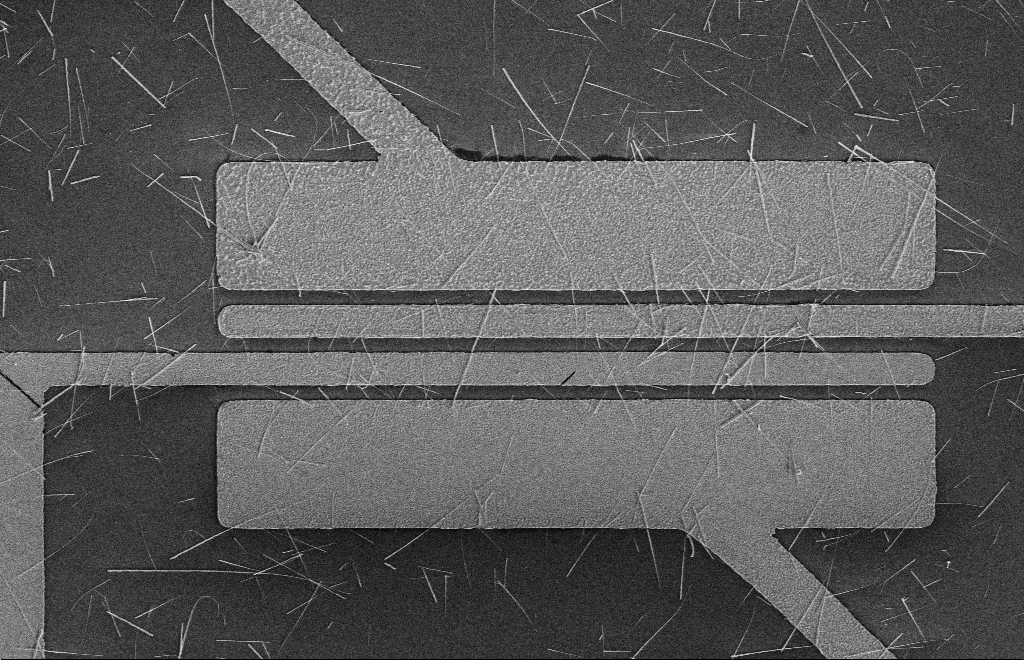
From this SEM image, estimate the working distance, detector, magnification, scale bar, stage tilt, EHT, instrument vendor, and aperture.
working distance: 16 mm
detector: SE2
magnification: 4.34 K X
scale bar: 10000 nm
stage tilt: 0°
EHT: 5 kV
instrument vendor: Zeiss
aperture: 10 µm